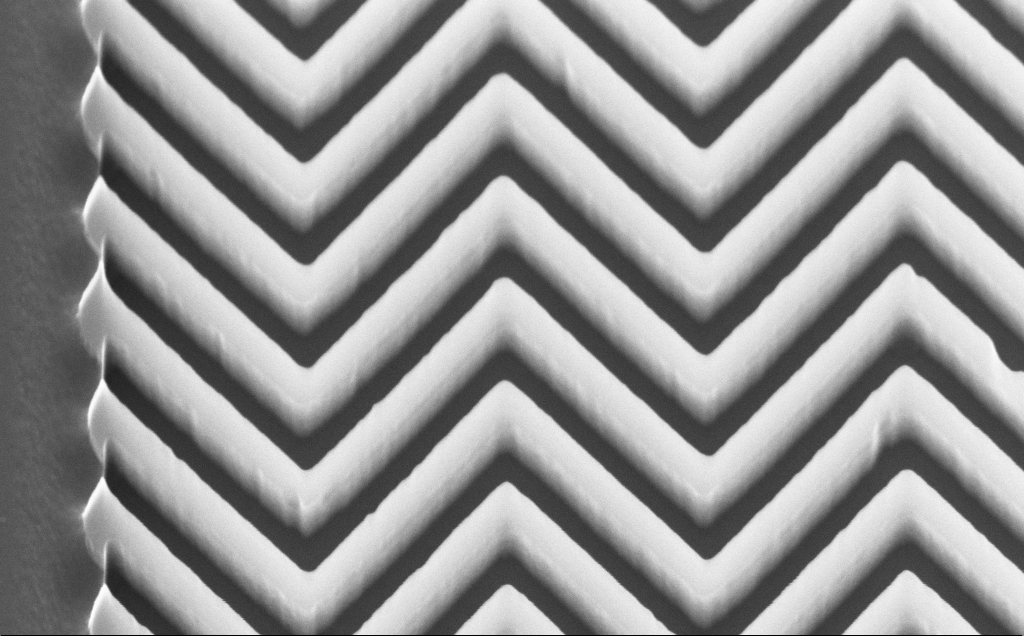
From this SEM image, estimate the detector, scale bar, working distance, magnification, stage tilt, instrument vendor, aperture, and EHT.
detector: InLens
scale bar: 200 nm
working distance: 4 mm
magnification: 73.89 K X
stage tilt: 30°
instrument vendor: Zeiss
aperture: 30 µm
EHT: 10 kV